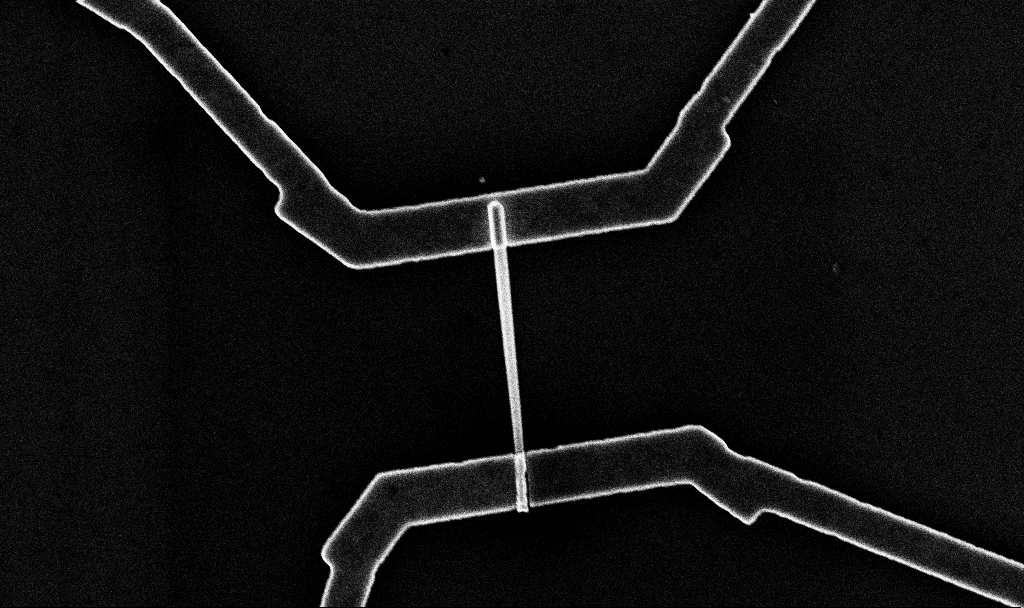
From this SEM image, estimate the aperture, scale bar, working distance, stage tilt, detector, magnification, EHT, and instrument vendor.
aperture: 30 µm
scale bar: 2000 nm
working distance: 6.7 mm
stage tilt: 0°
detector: InLens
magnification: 22.6 K X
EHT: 10 kV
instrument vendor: Zeiss